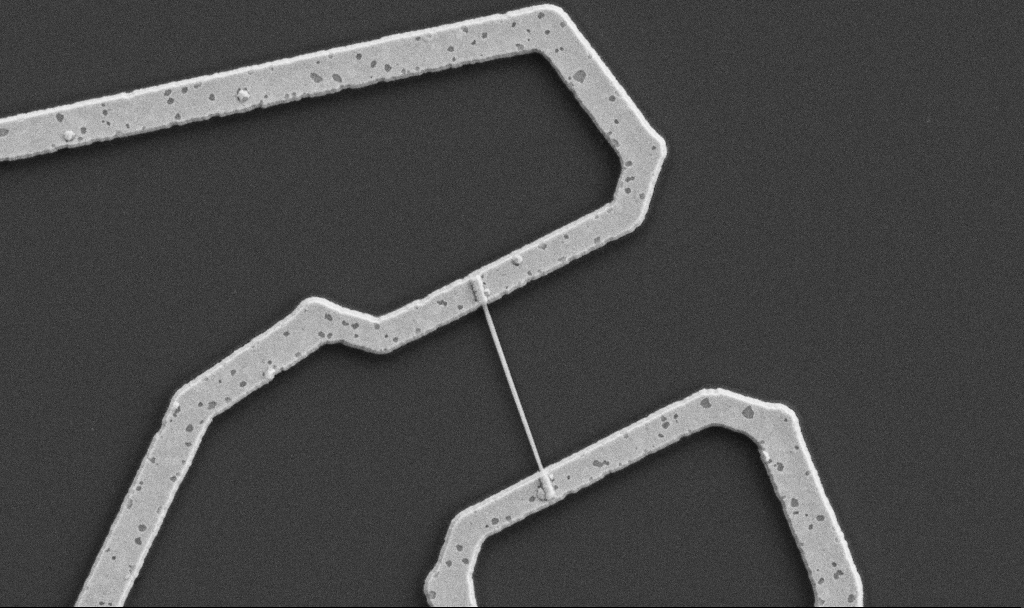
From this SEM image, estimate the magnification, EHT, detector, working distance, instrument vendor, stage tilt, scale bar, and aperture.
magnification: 20 K X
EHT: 5 kV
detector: SE2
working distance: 10.7 mm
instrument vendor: Zeiss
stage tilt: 0°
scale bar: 1000 nm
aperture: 30 µm